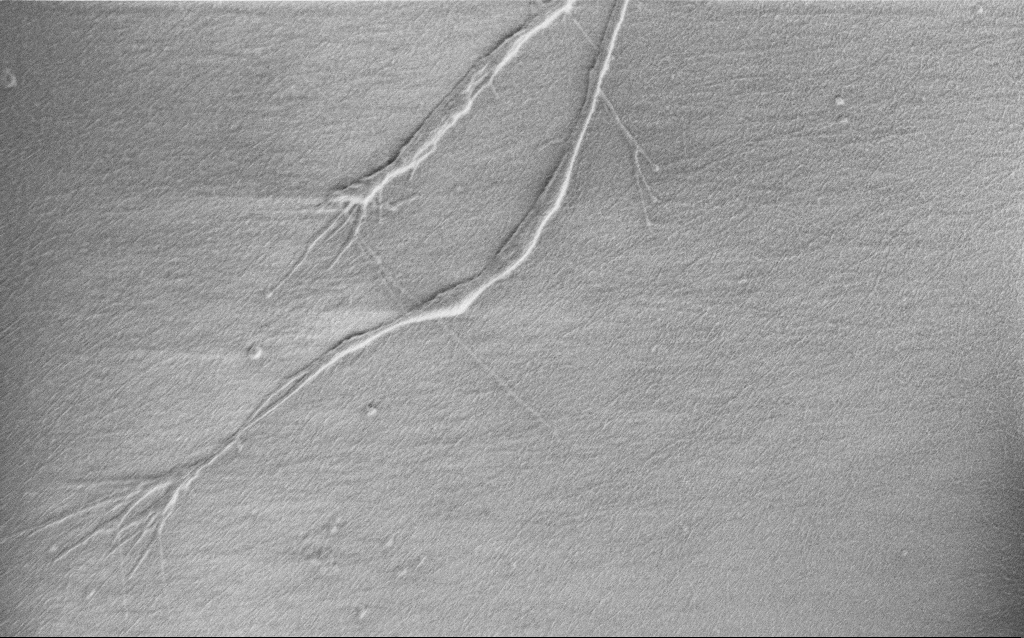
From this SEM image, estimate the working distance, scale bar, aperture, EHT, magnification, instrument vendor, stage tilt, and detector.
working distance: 6 mm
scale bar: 2000 nm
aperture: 30 µm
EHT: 0.9 kV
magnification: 7.5 K X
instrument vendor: Zeiss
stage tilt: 0°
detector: SE2